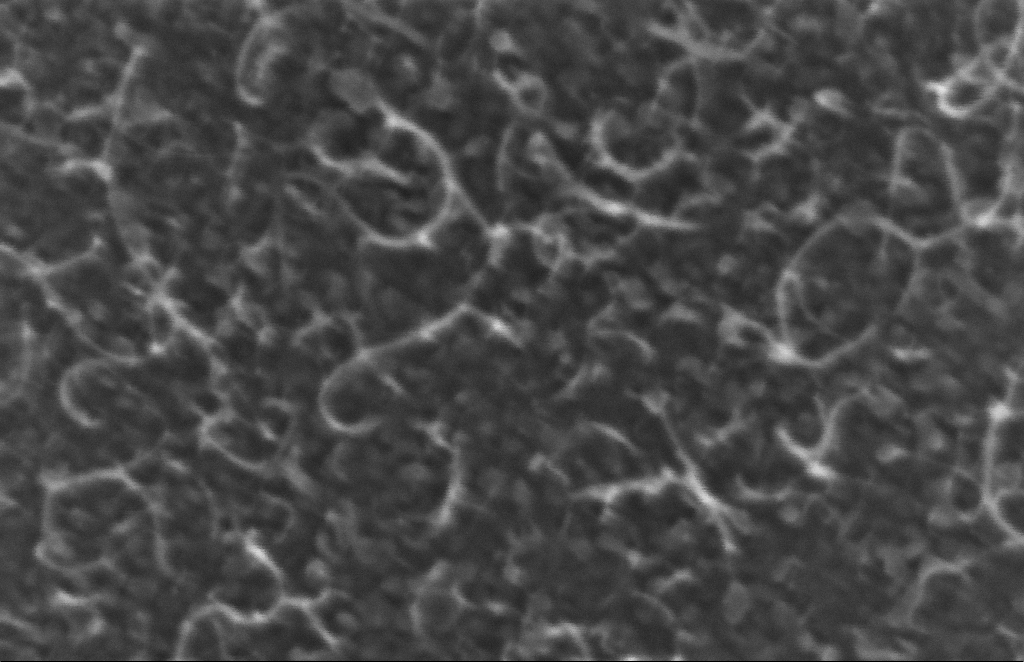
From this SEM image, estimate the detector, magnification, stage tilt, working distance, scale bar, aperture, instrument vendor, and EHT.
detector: InLens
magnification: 666.29 K X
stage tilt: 0°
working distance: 6 mm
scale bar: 20 nm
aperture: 30 µm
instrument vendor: Zeiss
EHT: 5 kV